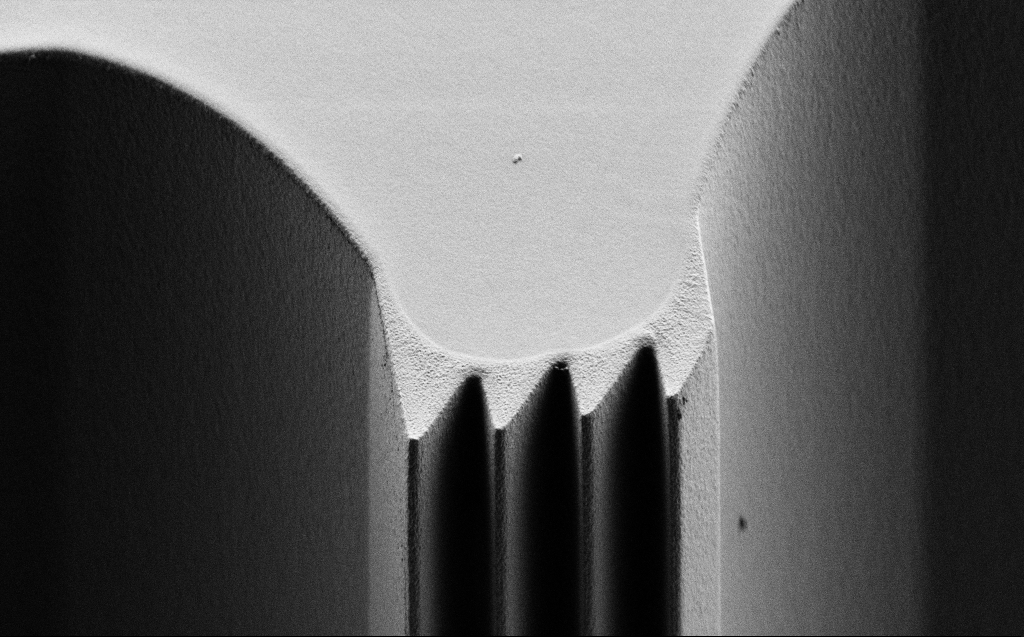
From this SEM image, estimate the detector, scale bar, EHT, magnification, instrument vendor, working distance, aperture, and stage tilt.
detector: SE2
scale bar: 10000 nm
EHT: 0.9 kV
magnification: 2.83 K X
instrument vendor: Zeiss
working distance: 6 mm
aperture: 30 µm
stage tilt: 45°